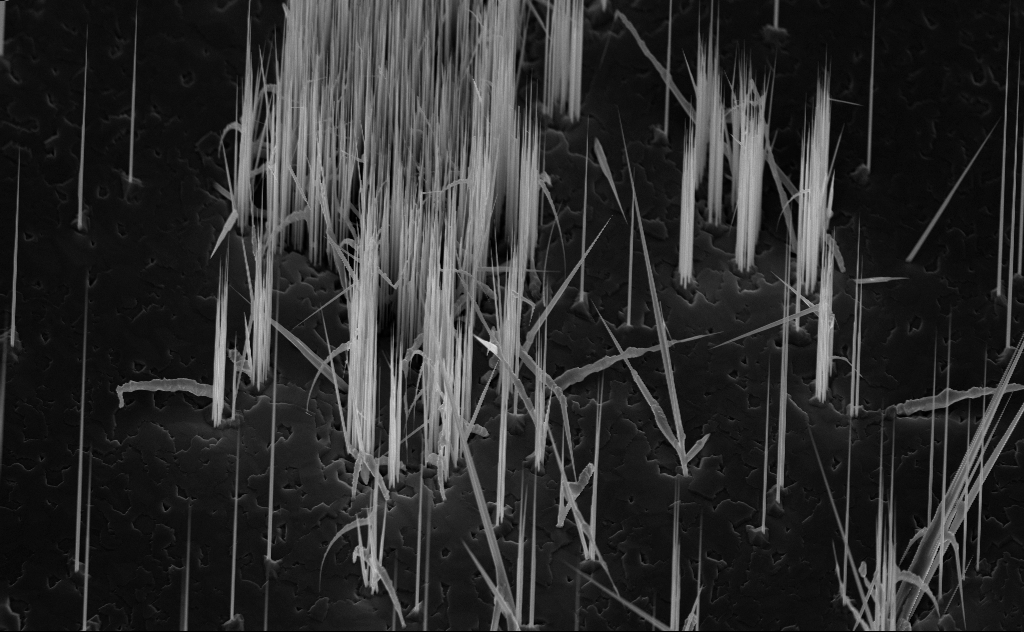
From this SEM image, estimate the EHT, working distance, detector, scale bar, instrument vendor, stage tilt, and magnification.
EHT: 10 kV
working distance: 7 mm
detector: InLens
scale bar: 10000 nm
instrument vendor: Zeiss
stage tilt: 45°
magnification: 6.23 K X